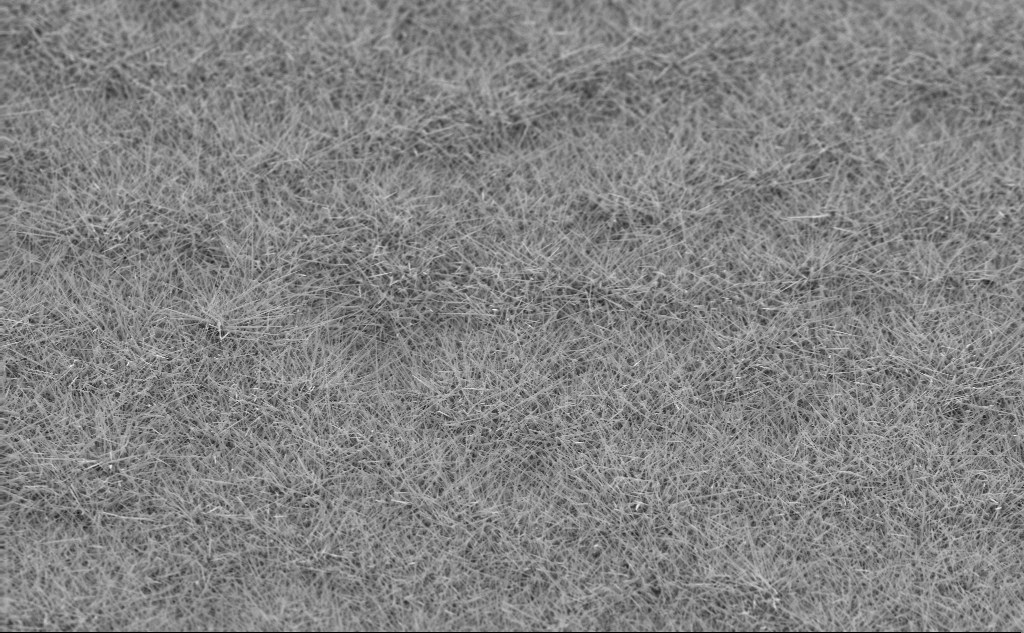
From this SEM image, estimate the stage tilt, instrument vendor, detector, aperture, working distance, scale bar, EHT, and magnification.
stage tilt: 45°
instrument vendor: Zeiss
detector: InLens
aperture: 30 µm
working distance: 4 mm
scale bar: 10000 nm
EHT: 10 kV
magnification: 5 K X